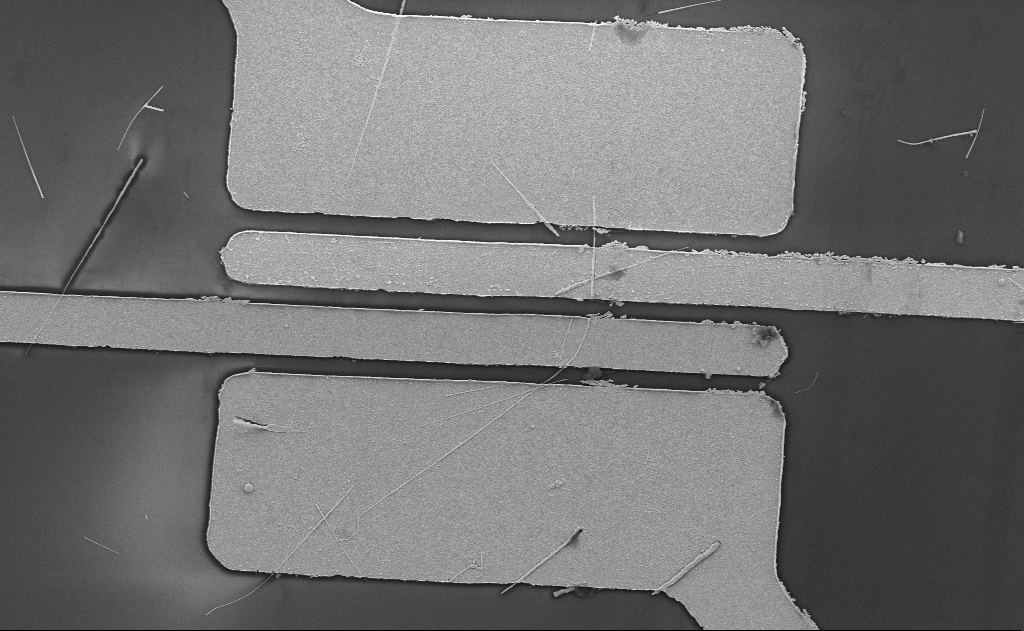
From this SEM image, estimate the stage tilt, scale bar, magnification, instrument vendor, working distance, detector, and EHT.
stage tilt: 0°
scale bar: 10000 nm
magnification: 6.72 K X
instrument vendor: Zeiss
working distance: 15 mm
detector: SE2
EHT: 5 kV